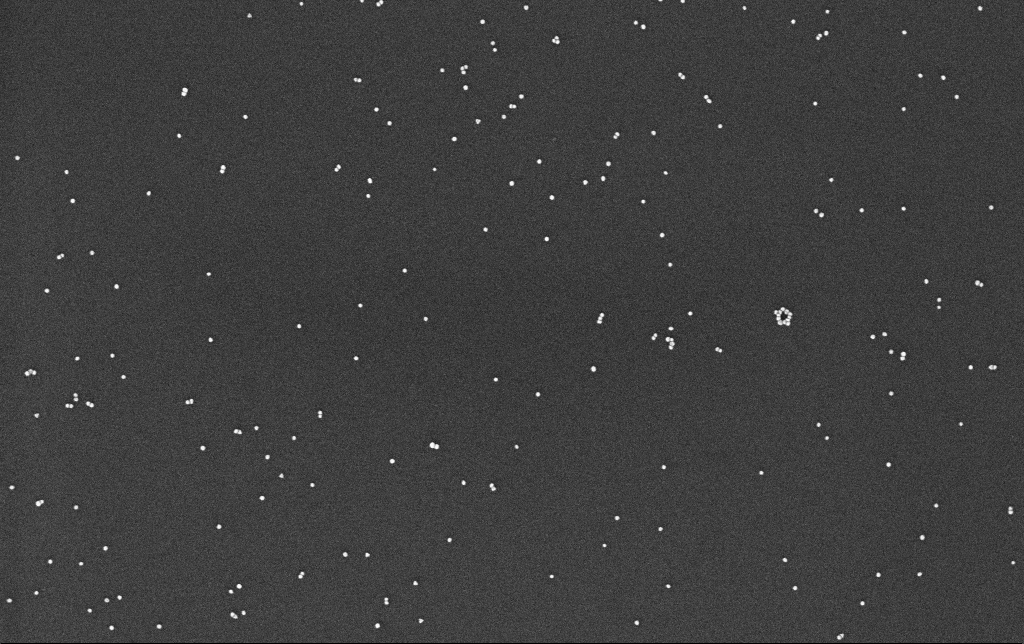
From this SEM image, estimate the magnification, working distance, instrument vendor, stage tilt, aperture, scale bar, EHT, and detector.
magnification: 100 K X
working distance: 3.3 mm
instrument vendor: Zeiss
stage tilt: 0°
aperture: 30 µm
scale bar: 200 nm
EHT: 10 kV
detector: InLens